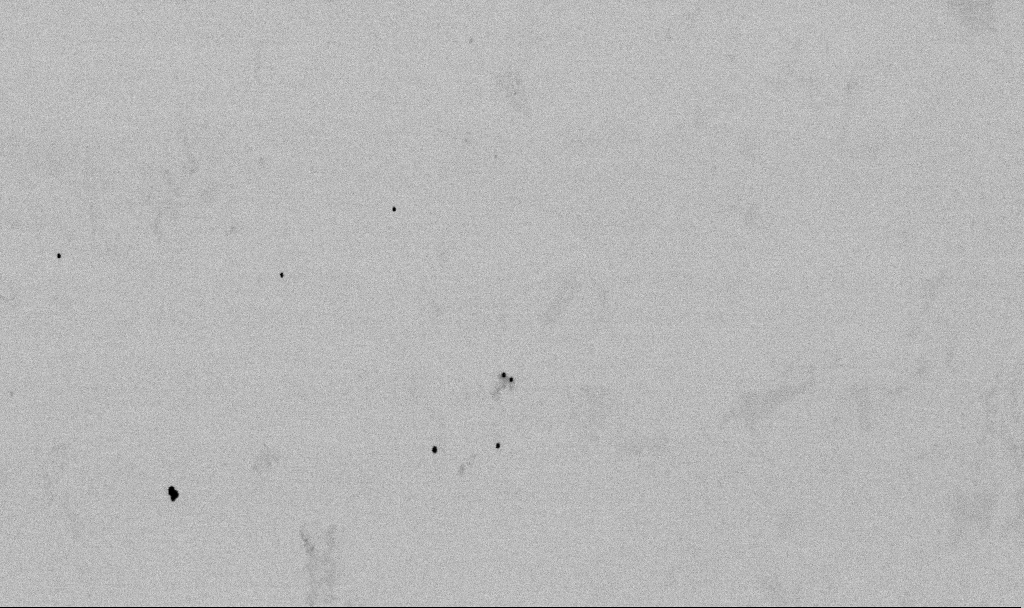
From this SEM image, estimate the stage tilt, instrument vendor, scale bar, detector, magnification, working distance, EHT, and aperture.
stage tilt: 0°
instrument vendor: Zeiss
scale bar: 1000 nm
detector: SE2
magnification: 50 K X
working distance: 6.5 mm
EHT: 2 kV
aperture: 30 µm